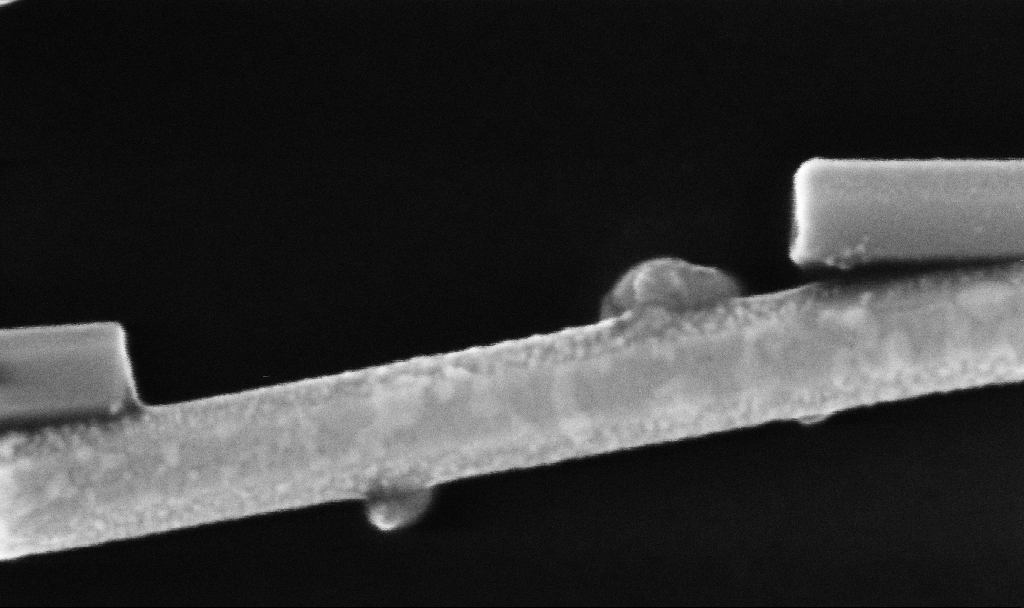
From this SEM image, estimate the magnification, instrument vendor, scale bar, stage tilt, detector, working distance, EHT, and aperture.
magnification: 369.35 K X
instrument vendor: Zeiss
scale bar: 100 nm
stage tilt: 0°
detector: InLens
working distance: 6.7 mm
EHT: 10 kV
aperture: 30 µm